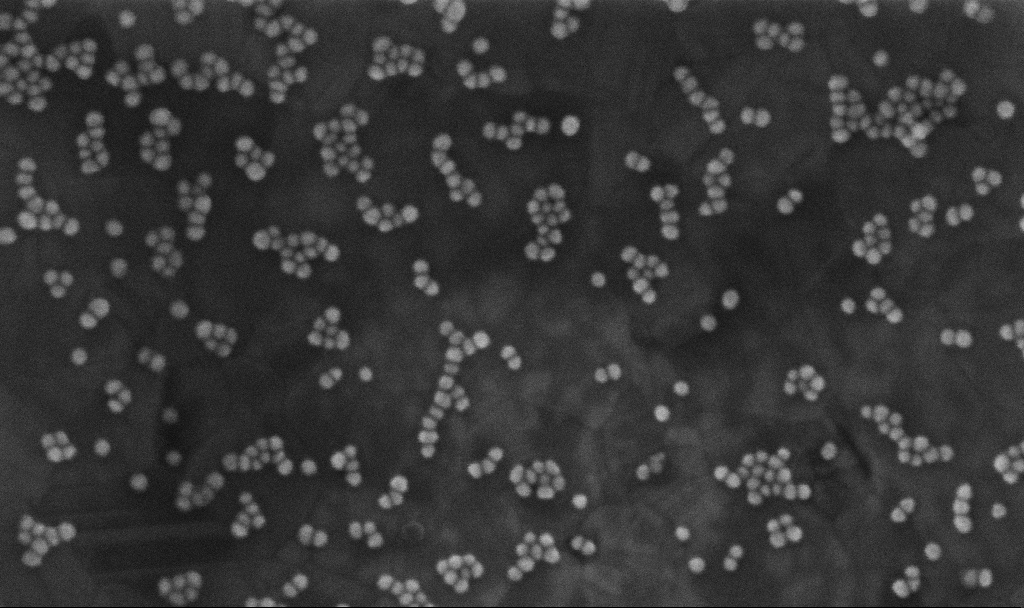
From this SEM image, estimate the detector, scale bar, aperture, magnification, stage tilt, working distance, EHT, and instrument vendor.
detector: InLens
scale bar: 200 nm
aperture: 30 µm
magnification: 300 K X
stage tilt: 0°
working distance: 3.8 mm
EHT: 10 kV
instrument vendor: Zeiss